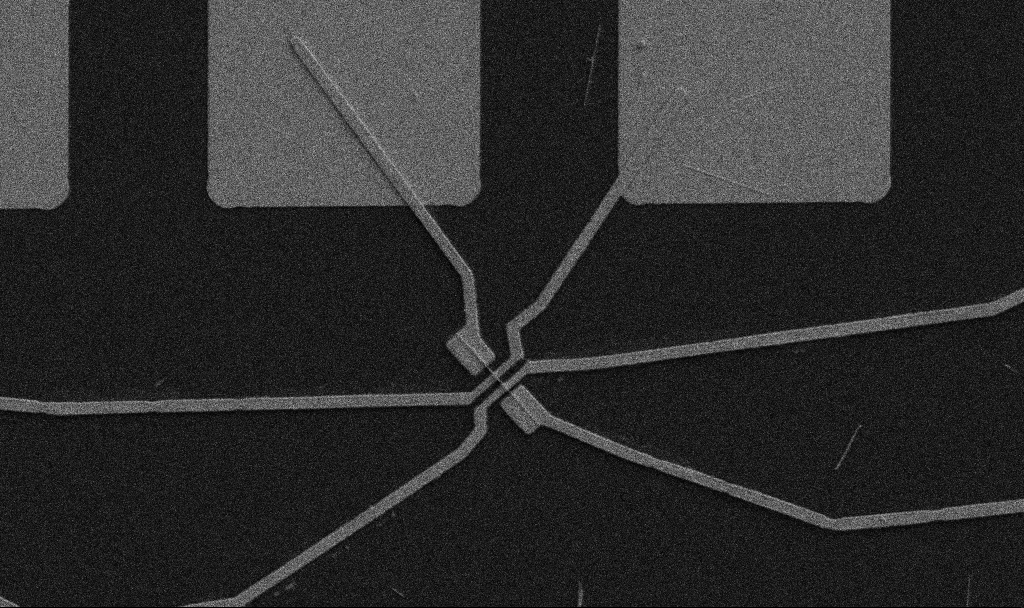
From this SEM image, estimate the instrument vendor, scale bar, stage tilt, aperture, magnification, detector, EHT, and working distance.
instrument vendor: Zeiss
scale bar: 10000 nm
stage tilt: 0°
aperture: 30 µm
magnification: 5 K X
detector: SE2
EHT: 5 kV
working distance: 10.7 mm